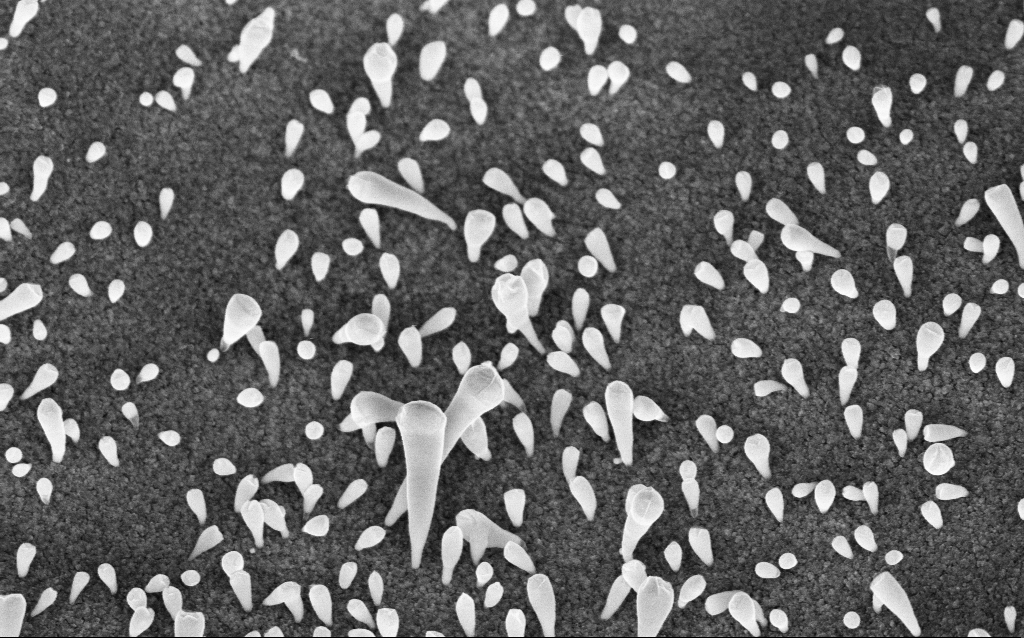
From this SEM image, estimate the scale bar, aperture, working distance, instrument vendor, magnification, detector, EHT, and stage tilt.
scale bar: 1000 nm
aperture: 30 µm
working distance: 6 mm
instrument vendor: Zeiss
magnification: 70.38 K X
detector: InLens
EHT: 5 kV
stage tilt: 42.9°